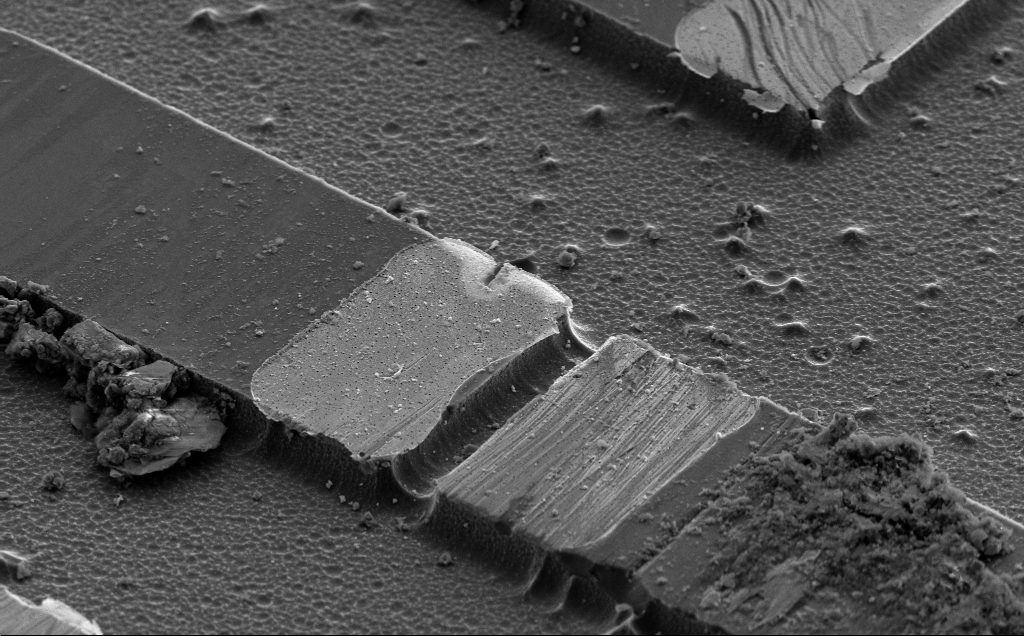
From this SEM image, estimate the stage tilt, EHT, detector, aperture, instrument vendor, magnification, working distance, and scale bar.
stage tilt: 50°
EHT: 5 kV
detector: SE2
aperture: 30 µm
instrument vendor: Zeiss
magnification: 6.41 K X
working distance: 10 mm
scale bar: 10000 nm